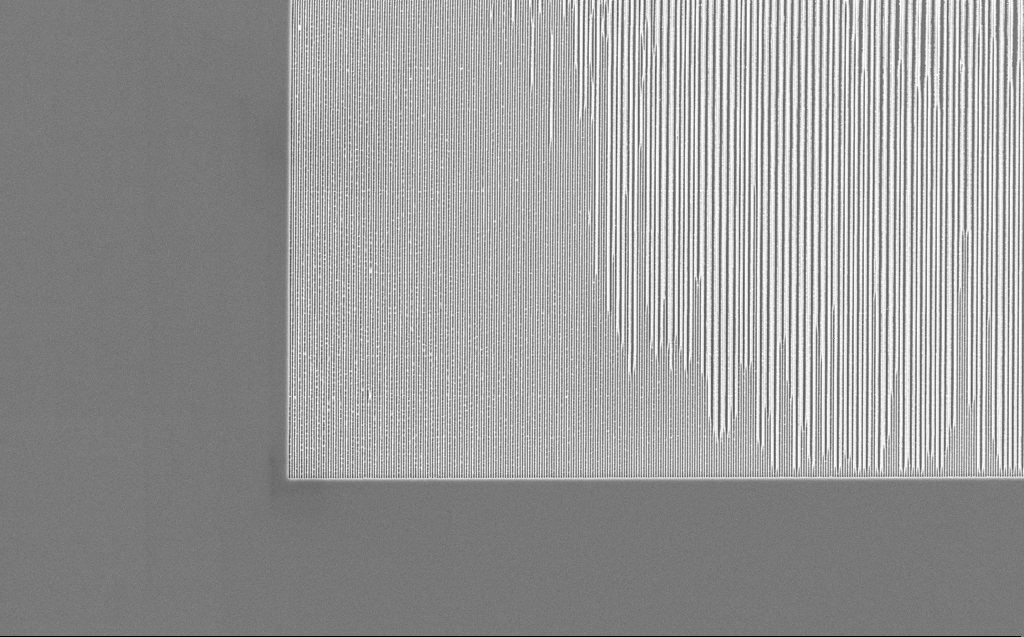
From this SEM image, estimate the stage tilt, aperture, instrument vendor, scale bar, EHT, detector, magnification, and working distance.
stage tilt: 0°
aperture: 30 µm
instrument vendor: Zeiss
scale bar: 10000 nm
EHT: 5 kV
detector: InLens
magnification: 5.95 K X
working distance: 7 mm